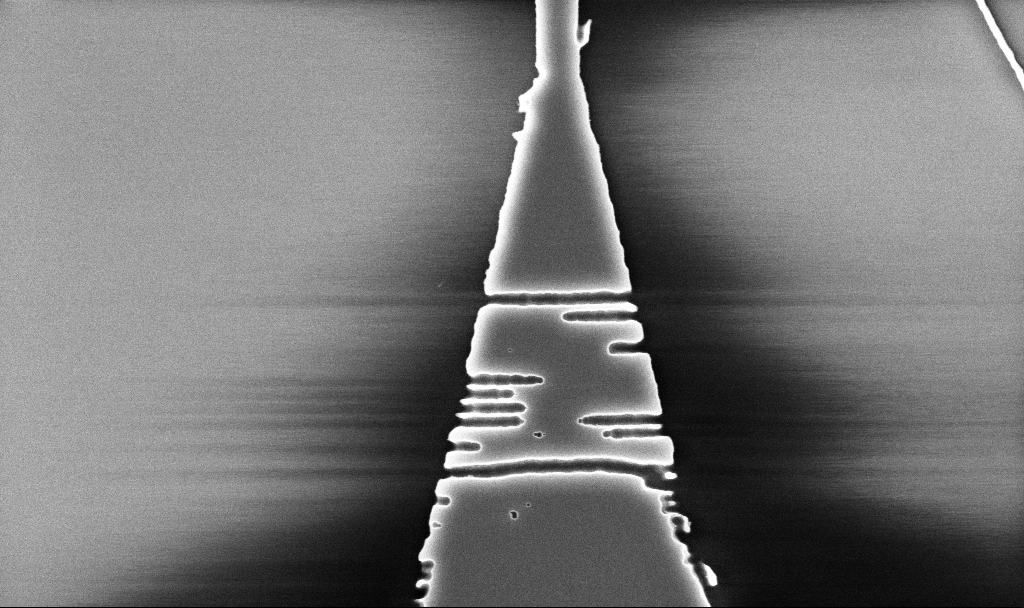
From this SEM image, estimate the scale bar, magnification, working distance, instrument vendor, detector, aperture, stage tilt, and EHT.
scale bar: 2000 nm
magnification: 31.32 K X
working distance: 5.2 mm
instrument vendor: Zeiss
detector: InLens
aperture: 30 µm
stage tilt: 0°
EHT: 5 kV